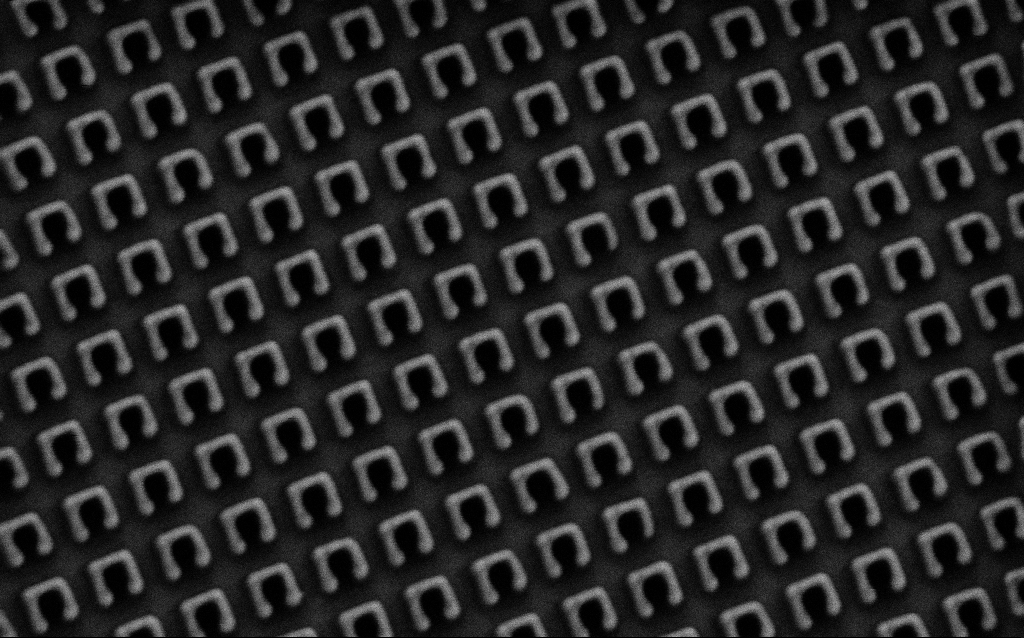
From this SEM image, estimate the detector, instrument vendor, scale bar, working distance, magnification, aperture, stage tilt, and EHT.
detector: SE2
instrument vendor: Zeiss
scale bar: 1000 nm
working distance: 7.9 mm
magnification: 55.73 K X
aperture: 30 µm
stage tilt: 0°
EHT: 1.5 kV